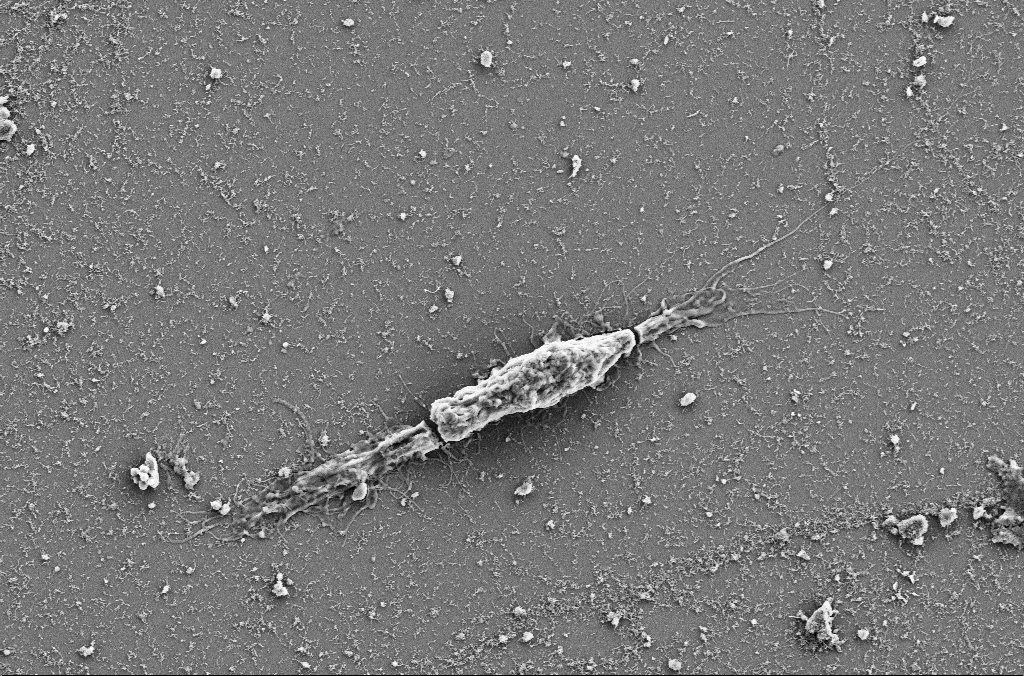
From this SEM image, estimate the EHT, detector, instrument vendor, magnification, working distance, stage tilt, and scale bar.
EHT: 5 kV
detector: SE2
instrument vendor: Zeiss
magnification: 4 K X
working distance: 4 mm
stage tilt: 0°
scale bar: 10000 nm